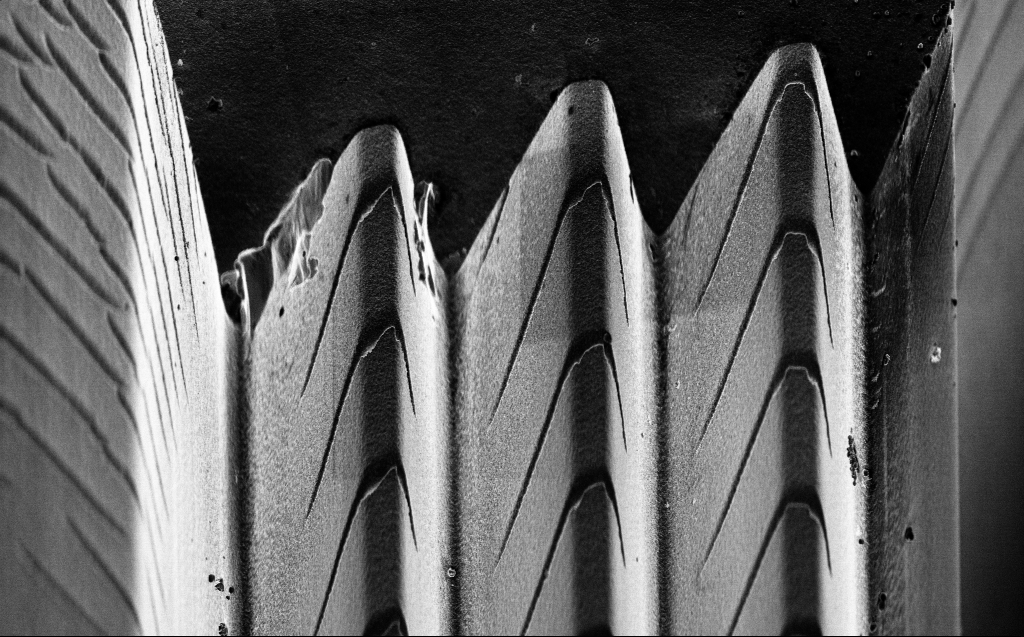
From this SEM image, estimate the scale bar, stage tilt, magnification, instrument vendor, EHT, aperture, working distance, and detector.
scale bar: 10000 nm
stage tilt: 45°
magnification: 6.97 K X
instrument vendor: Zeiss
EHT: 3 kV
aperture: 30 µm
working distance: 4 mm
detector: InLens